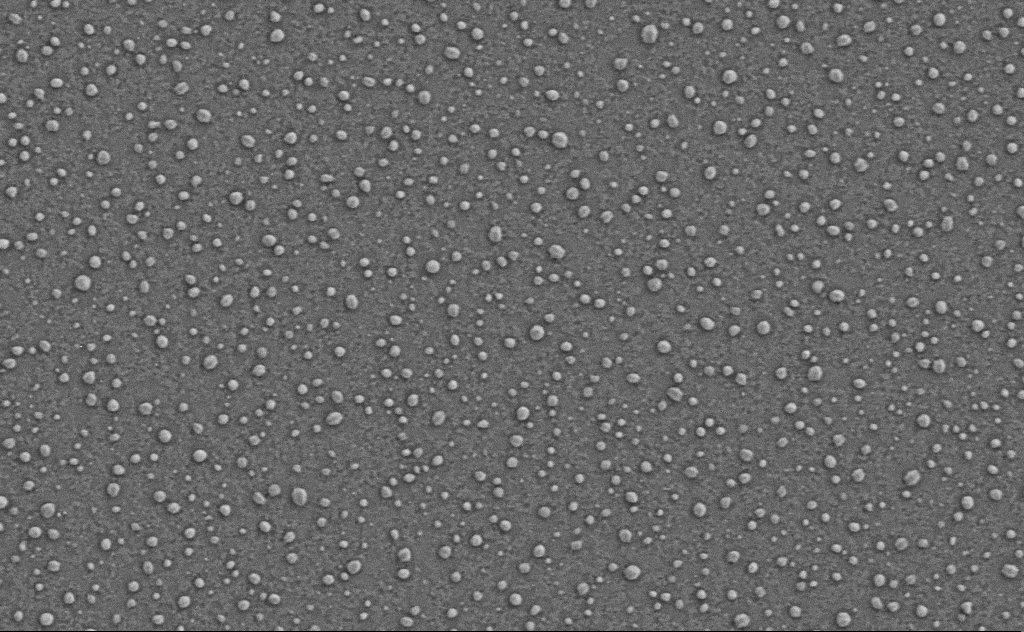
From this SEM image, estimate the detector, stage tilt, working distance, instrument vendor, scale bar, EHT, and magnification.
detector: SE2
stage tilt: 0°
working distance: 4 mm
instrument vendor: Zeiss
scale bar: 1000 nm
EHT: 3 kV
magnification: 40 K X